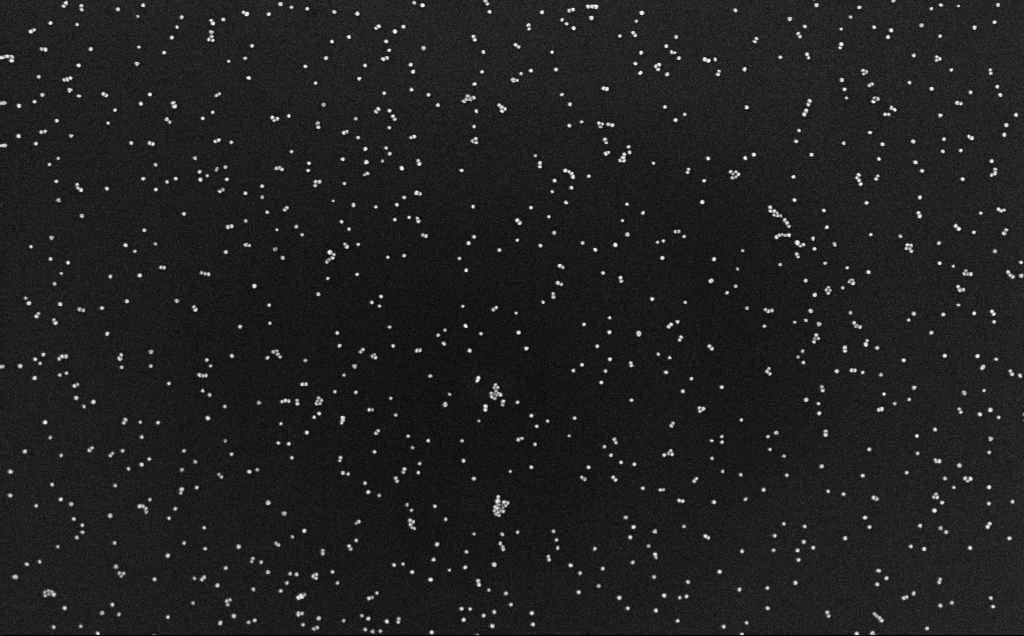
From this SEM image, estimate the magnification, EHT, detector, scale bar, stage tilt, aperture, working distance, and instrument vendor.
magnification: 100 K X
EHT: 10 kV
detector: InLens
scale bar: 200 nm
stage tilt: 0°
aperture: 30 µm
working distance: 3.1 mm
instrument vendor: Zeiss